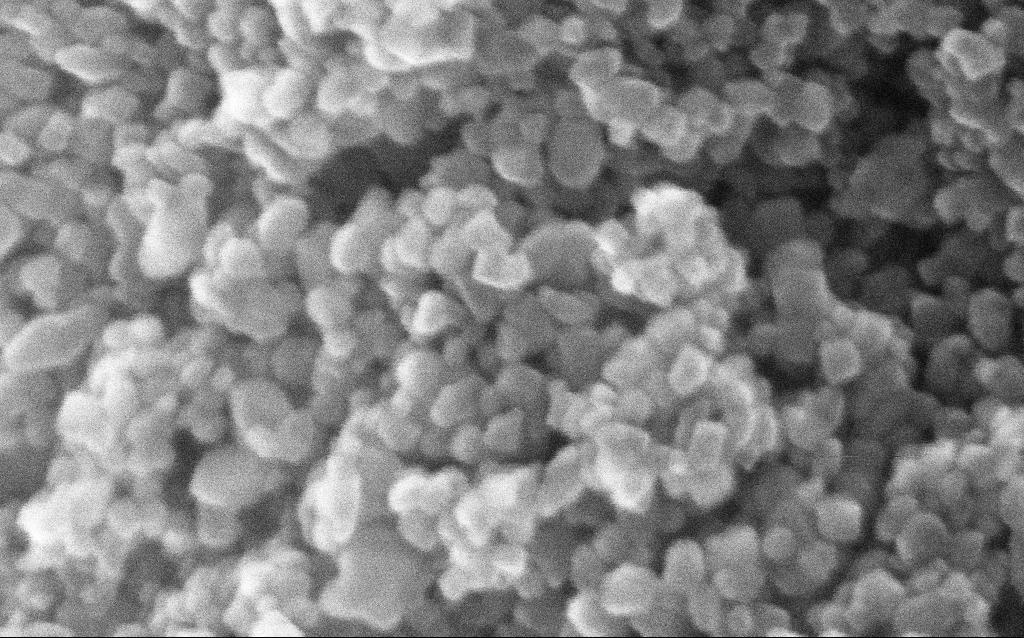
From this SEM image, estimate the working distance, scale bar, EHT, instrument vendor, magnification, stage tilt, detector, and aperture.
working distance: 1.8 mm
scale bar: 100 nm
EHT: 10 kV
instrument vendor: Zeiss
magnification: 588.95 K X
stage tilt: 0°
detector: InLens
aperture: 30 µm